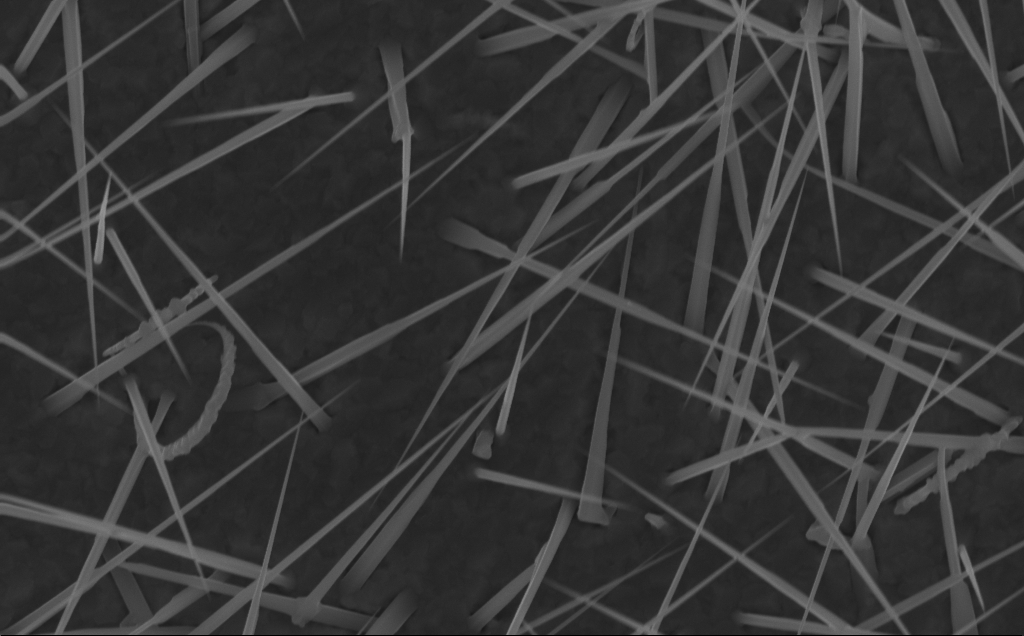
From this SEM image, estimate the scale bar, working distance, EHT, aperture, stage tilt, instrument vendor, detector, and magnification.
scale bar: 1000 nm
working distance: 6 mm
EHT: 10 kV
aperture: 30 µm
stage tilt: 0°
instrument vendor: Zeiss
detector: InLens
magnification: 40 K X